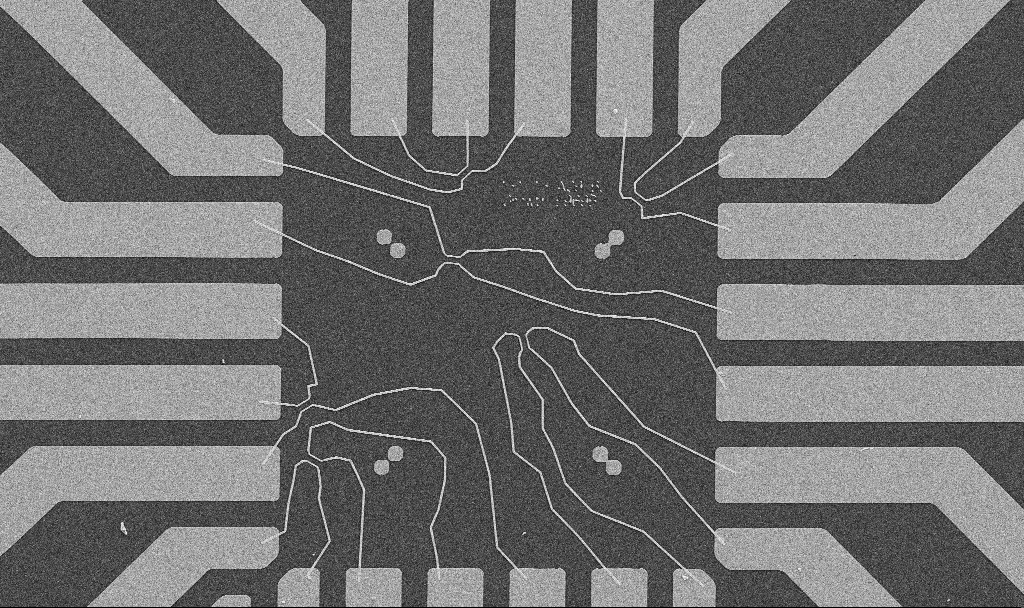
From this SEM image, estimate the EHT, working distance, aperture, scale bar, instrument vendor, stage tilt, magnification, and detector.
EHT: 10 kV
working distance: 10.7 mm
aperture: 30 µm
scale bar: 20000 nm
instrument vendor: Zeiss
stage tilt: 0°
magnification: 1 K X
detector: SE2